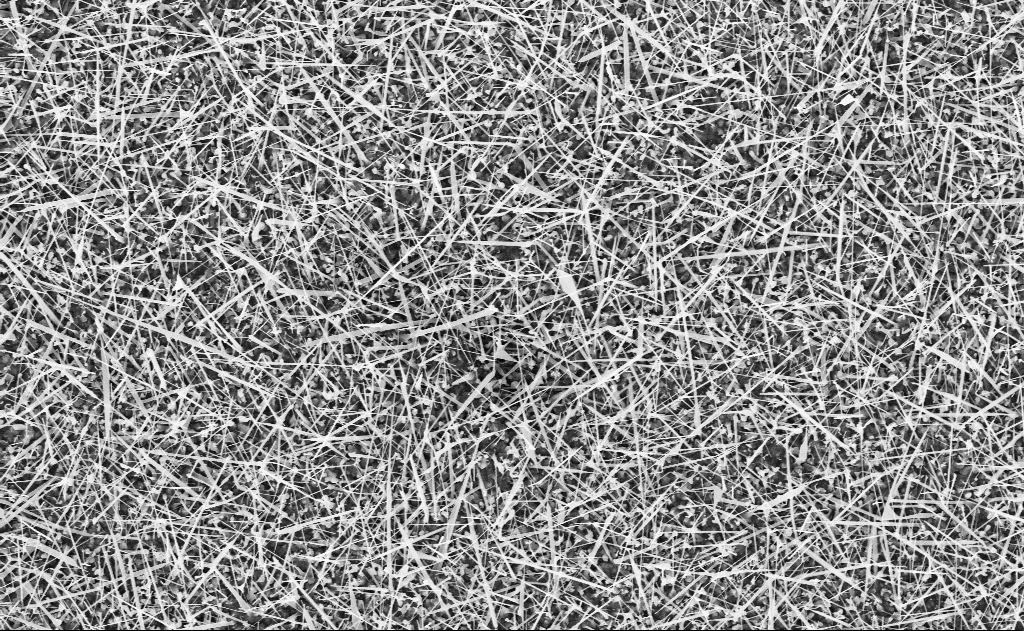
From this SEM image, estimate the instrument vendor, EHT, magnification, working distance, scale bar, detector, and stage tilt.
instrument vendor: Zeiss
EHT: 10 kV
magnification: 10 K X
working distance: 20 mm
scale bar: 2000 nm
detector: InLens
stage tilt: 0°